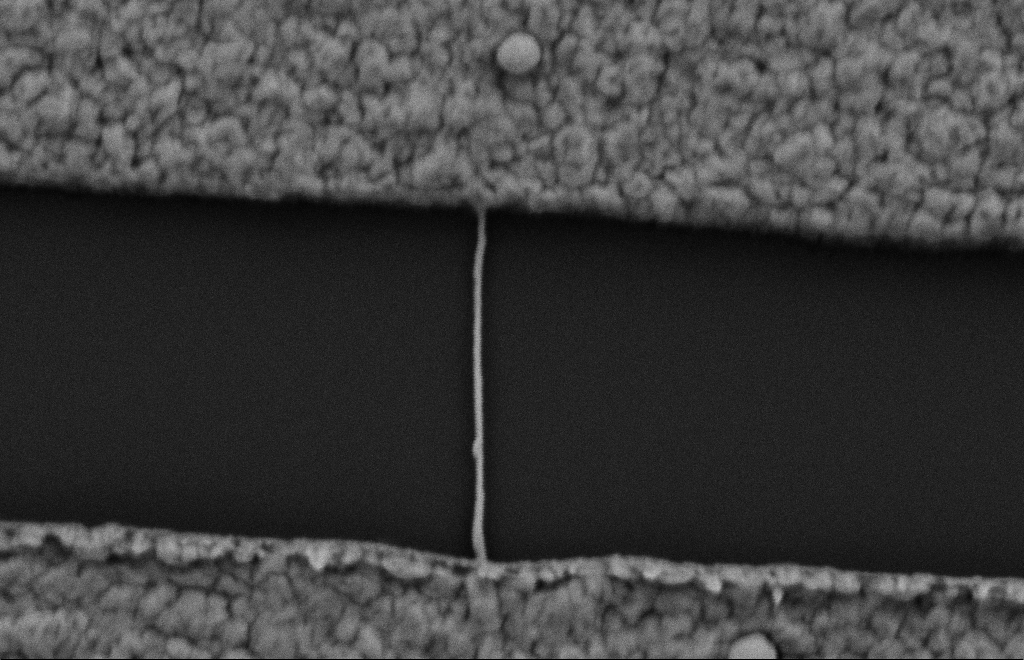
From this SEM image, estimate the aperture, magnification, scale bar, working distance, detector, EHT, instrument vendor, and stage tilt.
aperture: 20 µm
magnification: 89.4 K X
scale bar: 200 nm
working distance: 10 mm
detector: SE2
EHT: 2 kV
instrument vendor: Zeiss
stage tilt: -0.3°